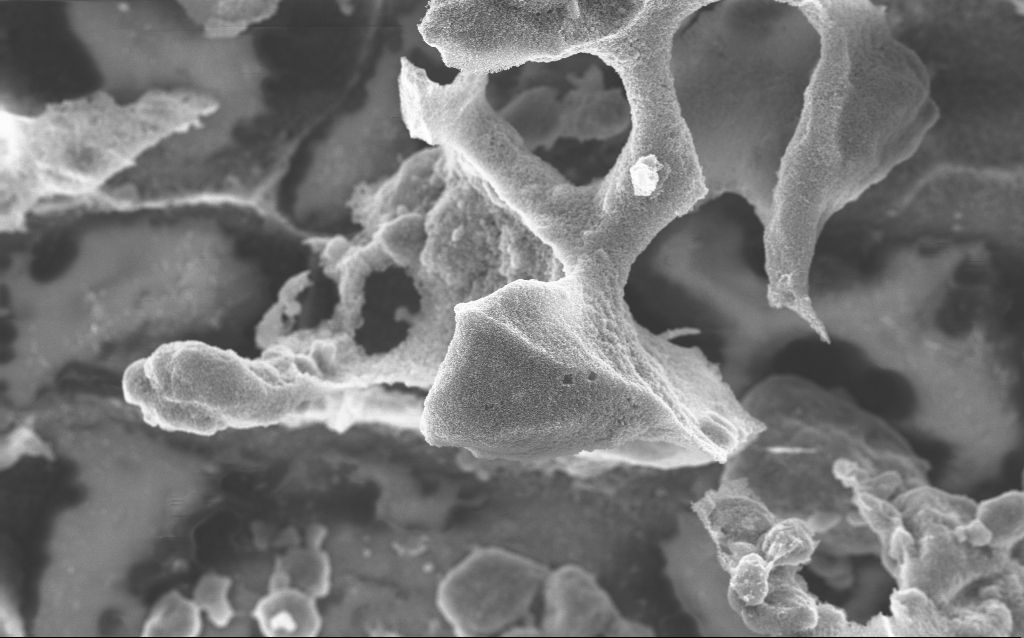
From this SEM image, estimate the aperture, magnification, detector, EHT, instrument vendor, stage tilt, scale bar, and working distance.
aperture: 30 µm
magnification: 5.83 K X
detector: InLens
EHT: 10 kV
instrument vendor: Zeiss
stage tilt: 0°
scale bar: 10000 nm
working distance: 2.7 mm